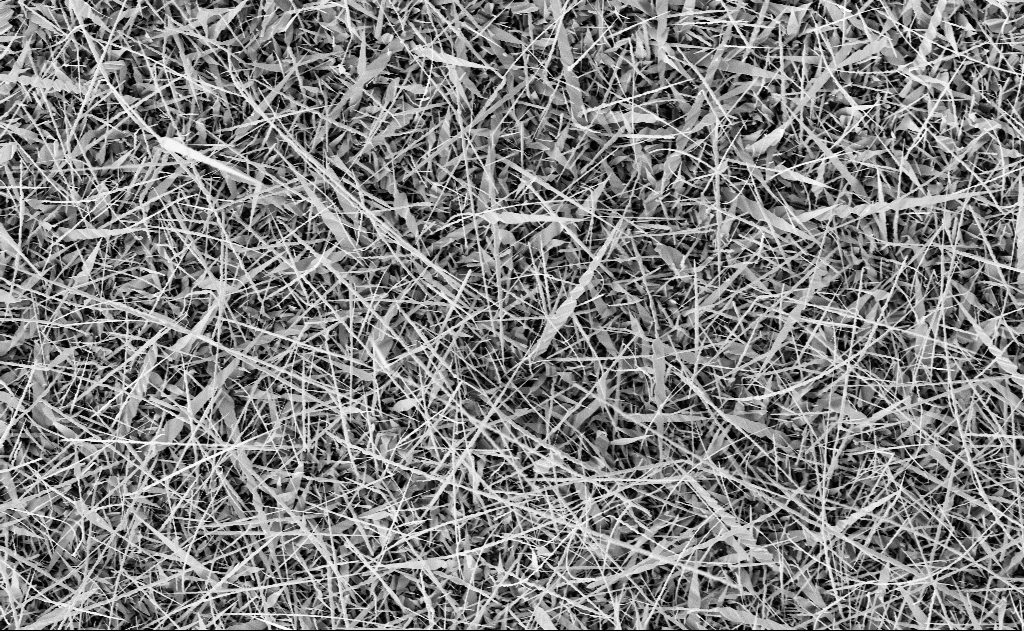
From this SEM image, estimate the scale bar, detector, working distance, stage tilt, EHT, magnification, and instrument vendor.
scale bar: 10000 nm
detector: InLens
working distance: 19 mm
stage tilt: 0°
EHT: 10 kV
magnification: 5 K X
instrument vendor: Zeiss